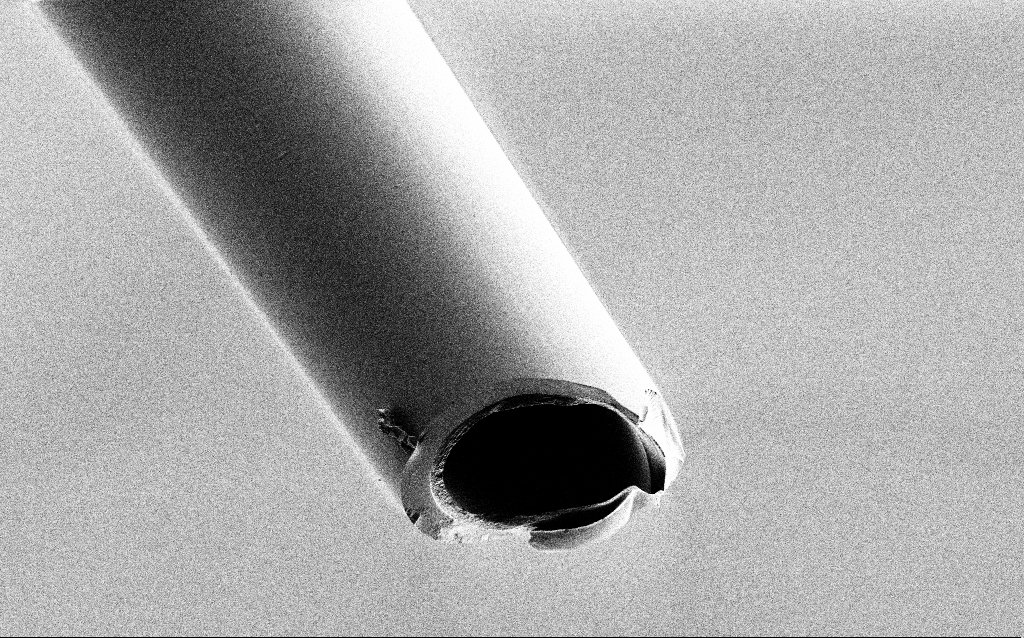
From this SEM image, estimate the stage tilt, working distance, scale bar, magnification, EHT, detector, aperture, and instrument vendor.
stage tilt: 45°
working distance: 7.5 mm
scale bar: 10000 nm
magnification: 2.5 K X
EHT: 1 kV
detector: SE2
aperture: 30 µm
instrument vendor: Zeiss